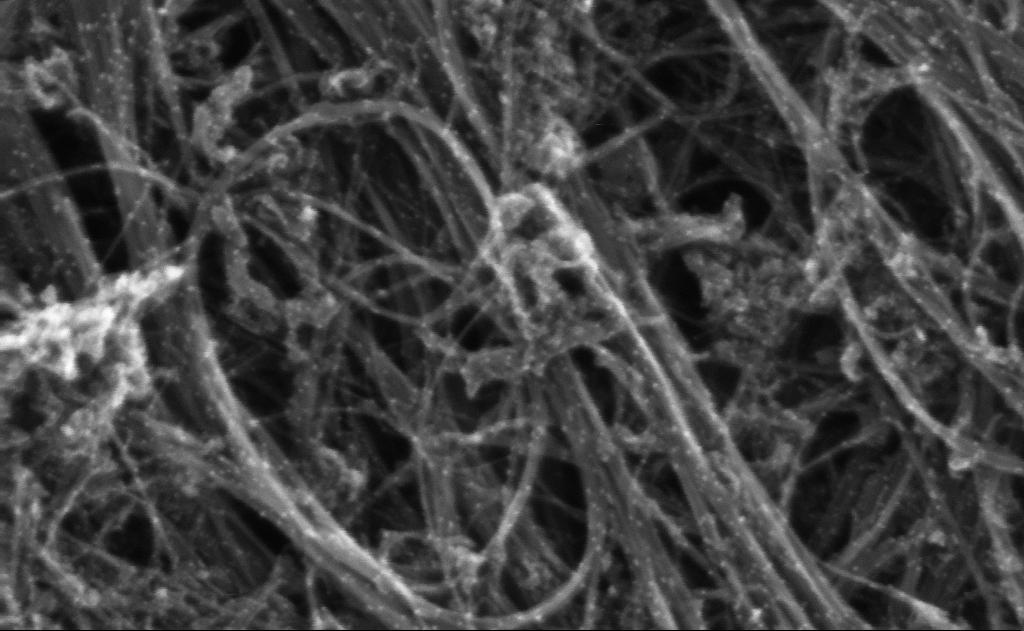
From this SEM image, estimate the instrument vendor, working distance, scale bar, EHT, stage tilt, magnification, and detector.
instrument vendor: Zeiss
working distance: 3 mm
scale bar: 100 nm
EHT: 10 kV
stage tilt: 0°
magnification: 510.12 K X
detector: InLens